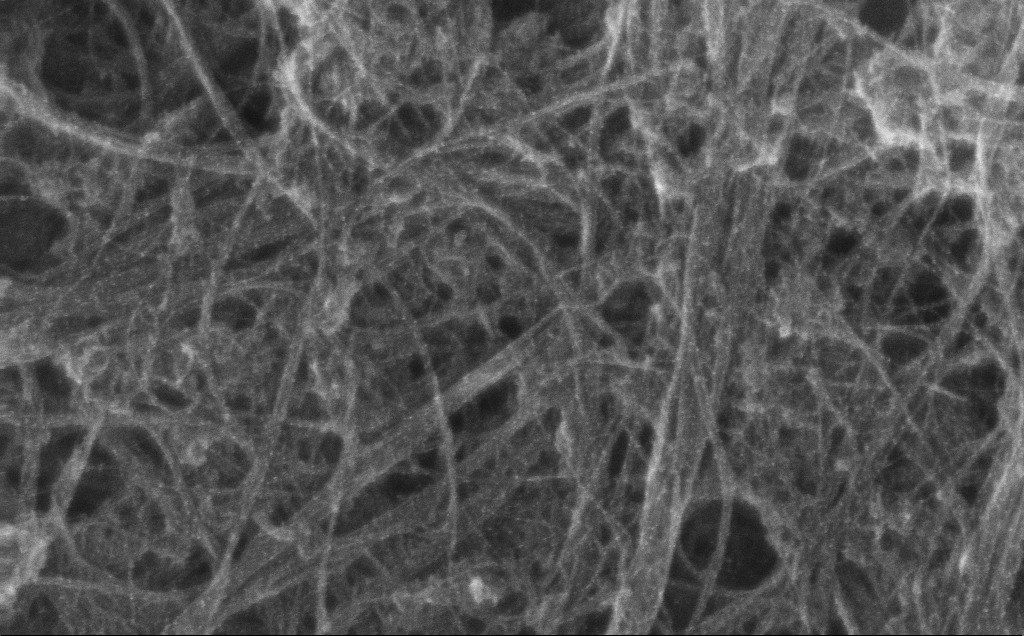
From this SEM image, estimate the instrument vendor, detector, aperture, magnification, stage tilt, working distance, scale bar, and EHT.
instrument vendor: Zeiss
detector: InLens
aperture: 30 µm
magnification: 174.07 K X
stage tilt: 0°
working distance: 3 mm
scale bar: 200 nm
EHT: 10 kV